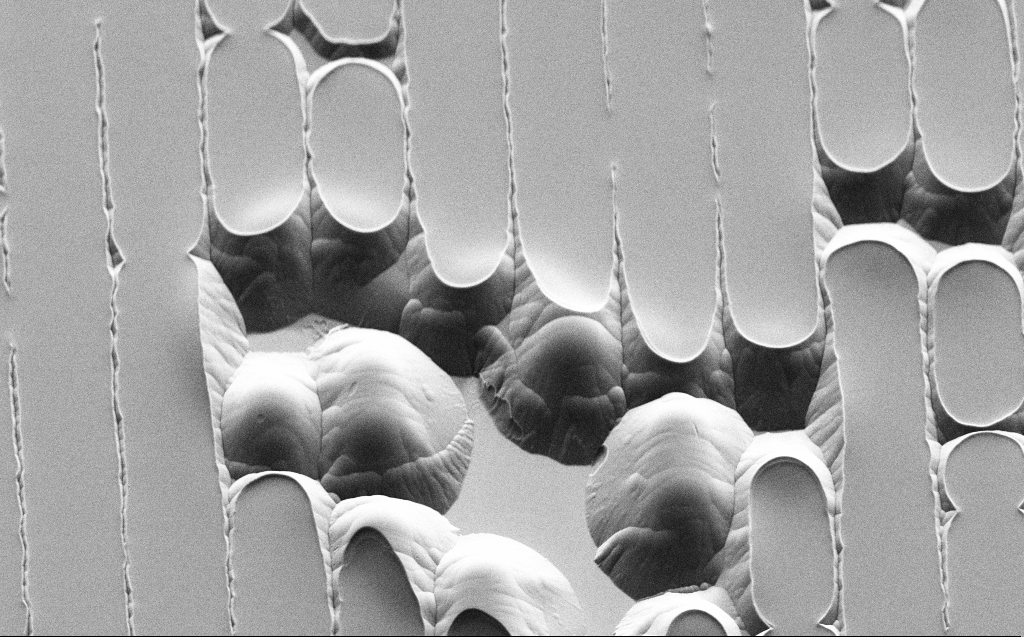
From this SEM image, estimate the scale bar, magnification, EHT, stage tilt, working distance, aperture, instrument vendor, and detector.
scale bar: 10000 nm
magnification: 3.73 K X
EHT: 3 kV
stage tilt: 45°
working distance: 9 mm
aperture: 30 µm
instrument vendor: Zeiss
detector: SE2